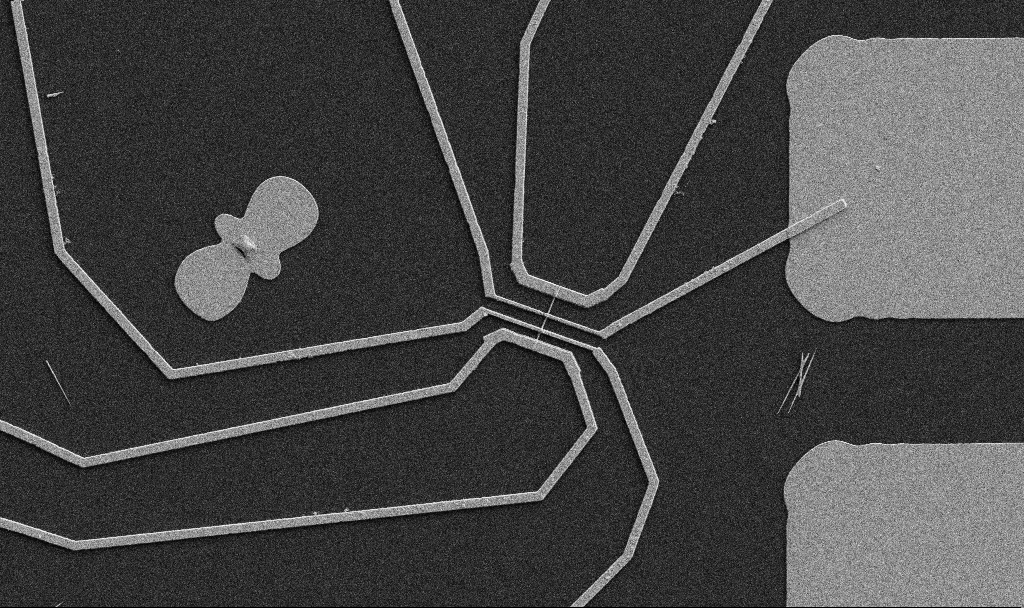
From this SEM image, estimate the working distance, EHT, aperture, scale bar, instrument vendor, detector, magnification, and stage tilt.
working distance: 10.7 mm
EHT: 5 kV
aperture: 30 µm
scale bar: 10000 nm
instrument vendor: Zeiss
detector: SE2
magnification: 5 K X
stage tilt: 0°